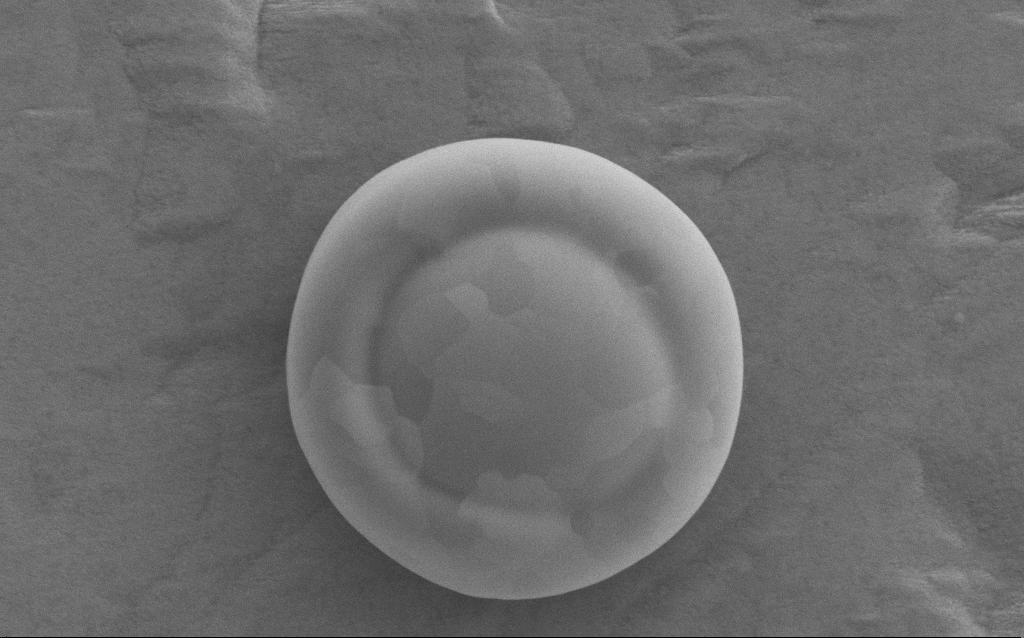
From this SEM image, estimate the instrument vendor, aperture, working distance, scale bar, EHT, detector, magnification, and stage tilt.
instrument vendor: Zeiss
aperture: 30 µm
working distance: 4 mm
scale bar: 1000 nm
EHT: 5 kV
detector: SE2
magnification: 40 K X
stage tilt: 0°